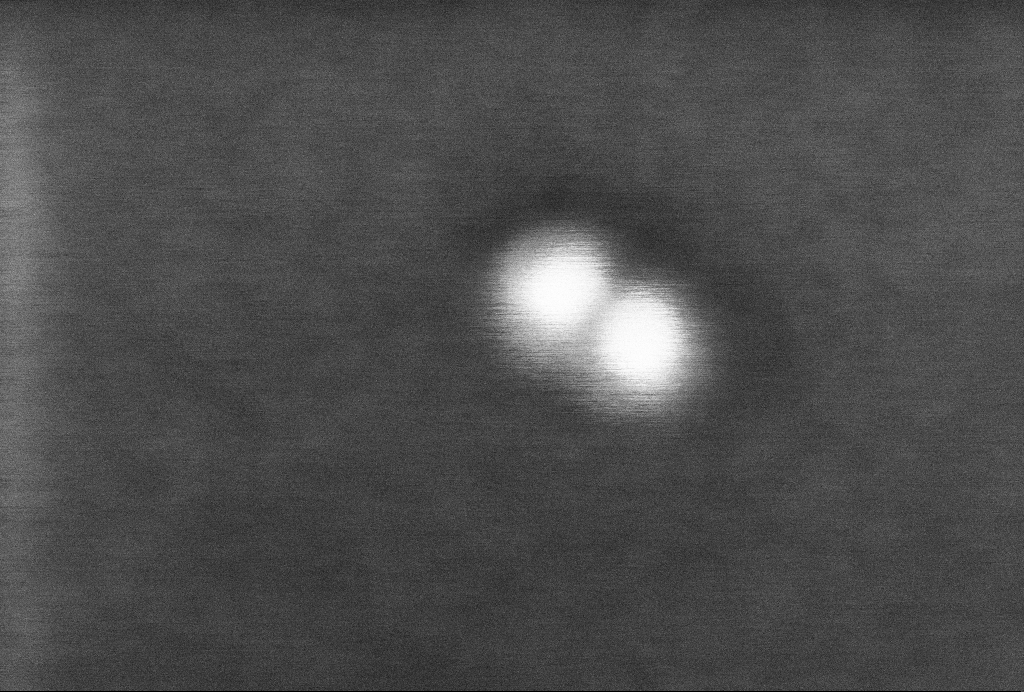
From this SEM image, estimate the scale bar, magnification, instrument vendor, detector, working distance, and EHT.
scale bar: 20 nm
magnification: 1454.24 K X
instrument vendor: Zeiss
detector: InLens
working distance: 3.3 mm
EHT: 2 kV